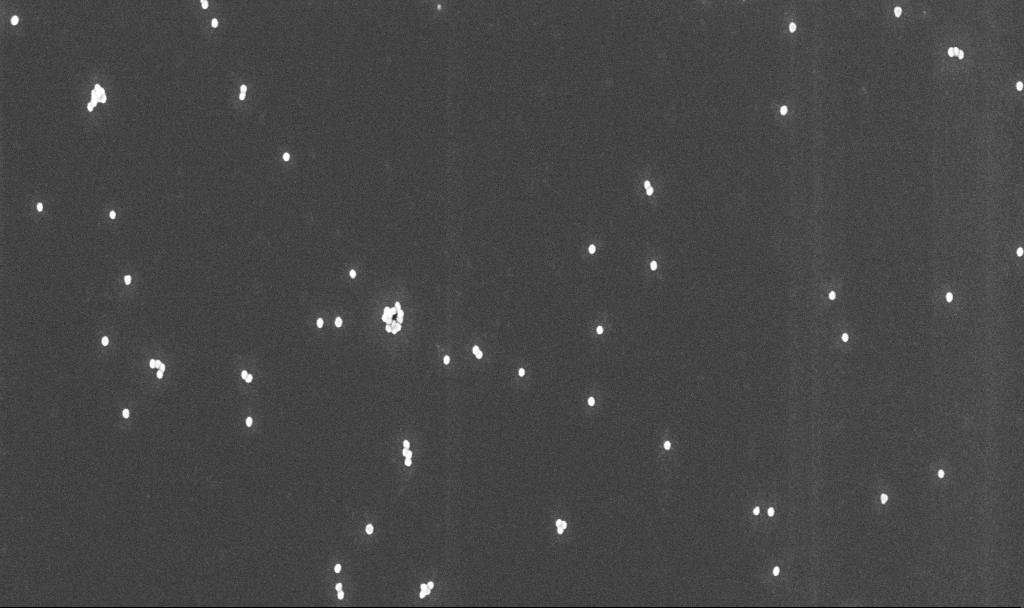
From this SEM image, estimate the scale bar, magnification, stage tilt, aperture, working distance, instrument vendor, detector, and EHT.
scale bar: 200 nm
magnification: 100 K X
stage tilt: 0°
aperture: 30 µm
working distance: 4.8 mm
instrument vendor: Zeiss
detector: InLens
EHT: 10 kV